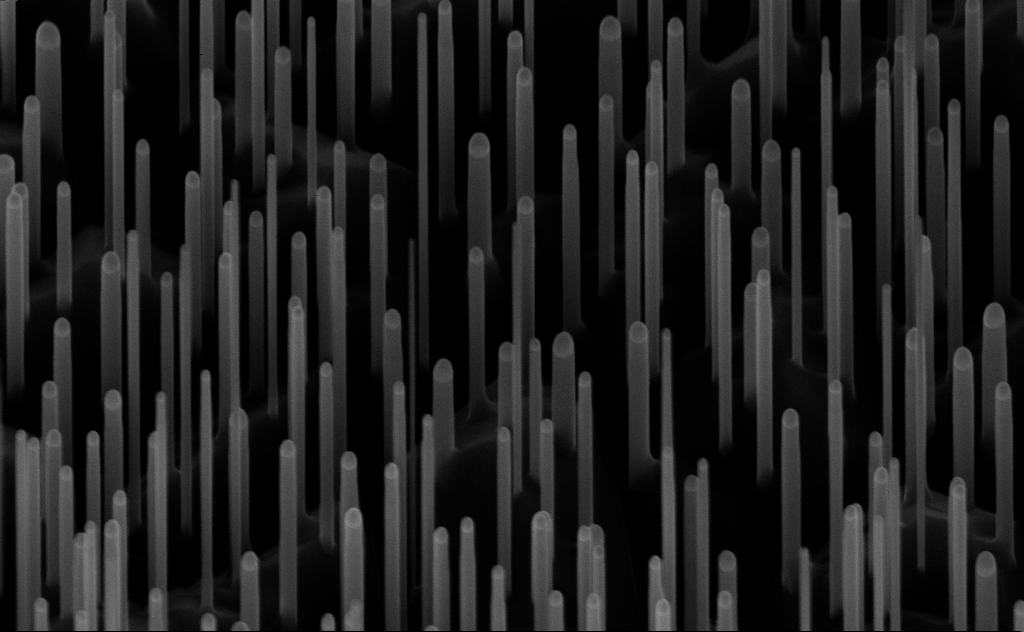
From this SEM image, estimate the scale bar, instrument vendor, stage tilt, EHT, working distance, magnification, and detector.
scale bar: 200 nm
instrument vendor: Zeiss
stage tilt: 45°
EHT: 10 kV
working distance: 7 mm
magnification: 80 K X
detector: InLens